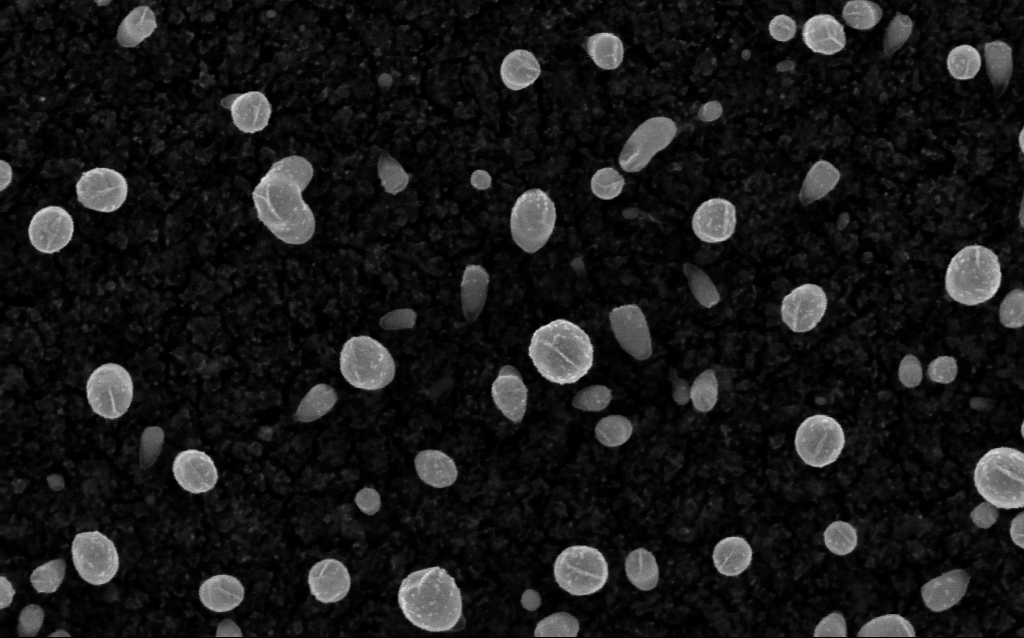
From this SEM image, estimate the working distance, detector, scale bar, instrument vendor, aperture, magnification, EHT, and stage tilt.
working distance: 2.1 mm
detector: InLens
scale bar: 100 nm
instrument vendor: Zeiss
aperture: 30 µm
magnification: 200 K X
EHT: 5 kV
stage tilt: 0°